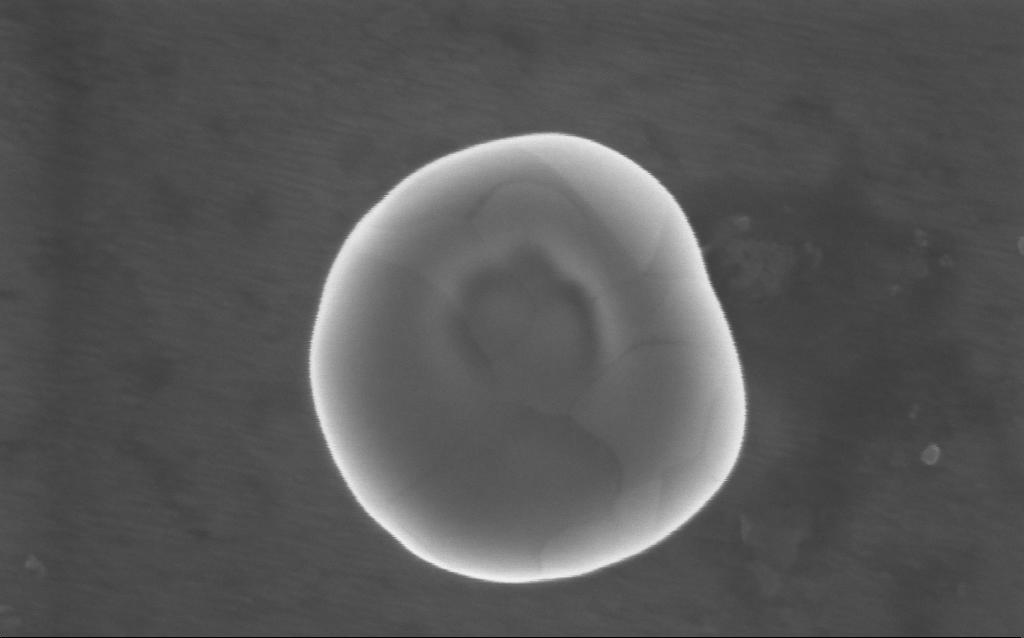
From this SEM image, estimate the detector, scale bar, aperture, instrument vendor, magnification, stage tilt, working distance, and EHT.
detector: InLens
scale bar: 200 nm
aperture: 30 µm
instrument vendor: Zeiss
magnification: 232 K X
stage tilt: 0°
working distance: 4 mm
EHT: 5 kV